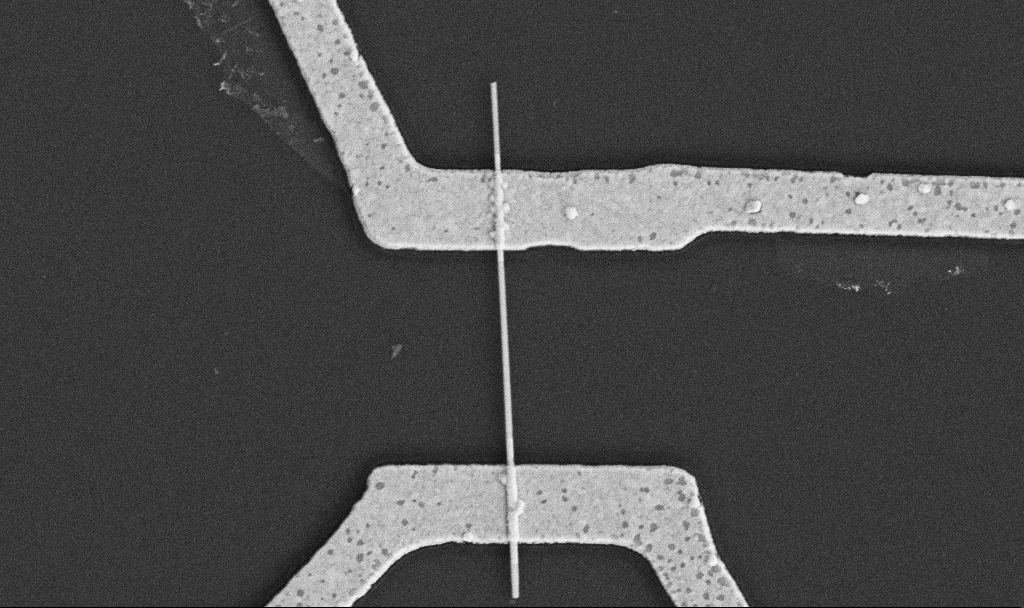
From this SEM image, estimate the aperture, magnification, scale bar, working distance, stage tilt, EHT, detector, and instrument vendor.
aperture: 30 µm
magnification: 30 K X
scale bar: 1000 nm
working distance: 10.6 mm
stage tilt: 0°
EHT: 5 kV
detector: SE2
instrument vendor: Zeiss